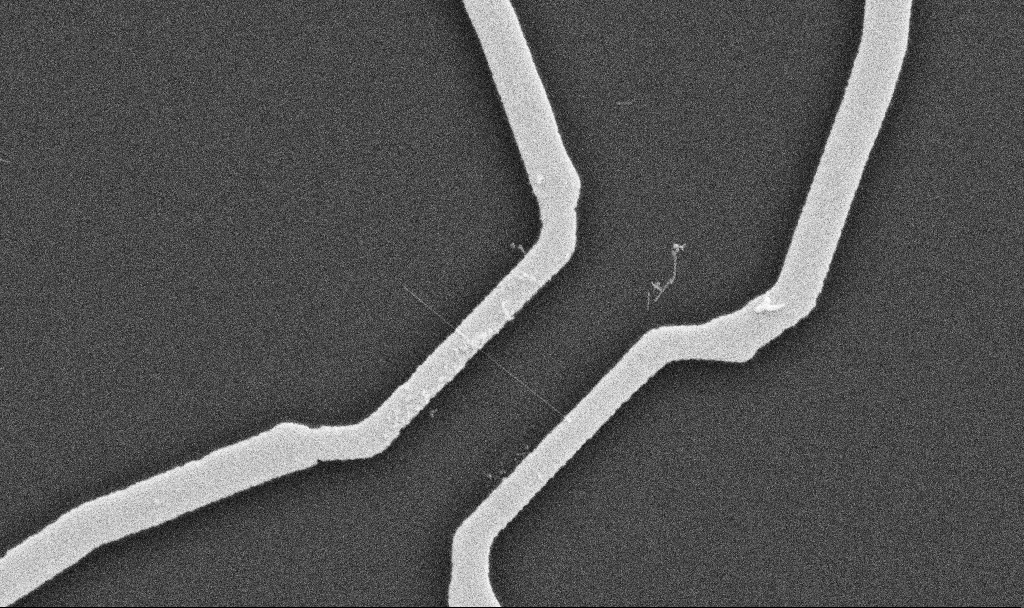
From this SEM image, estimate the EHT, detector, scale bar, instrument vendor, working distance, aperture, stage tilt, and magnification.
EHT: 10 kV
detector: SE2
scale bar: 1000 nm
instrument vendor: Zeiss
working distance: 10.7 mm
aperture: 30 µm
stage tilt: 0°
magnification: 20 K X